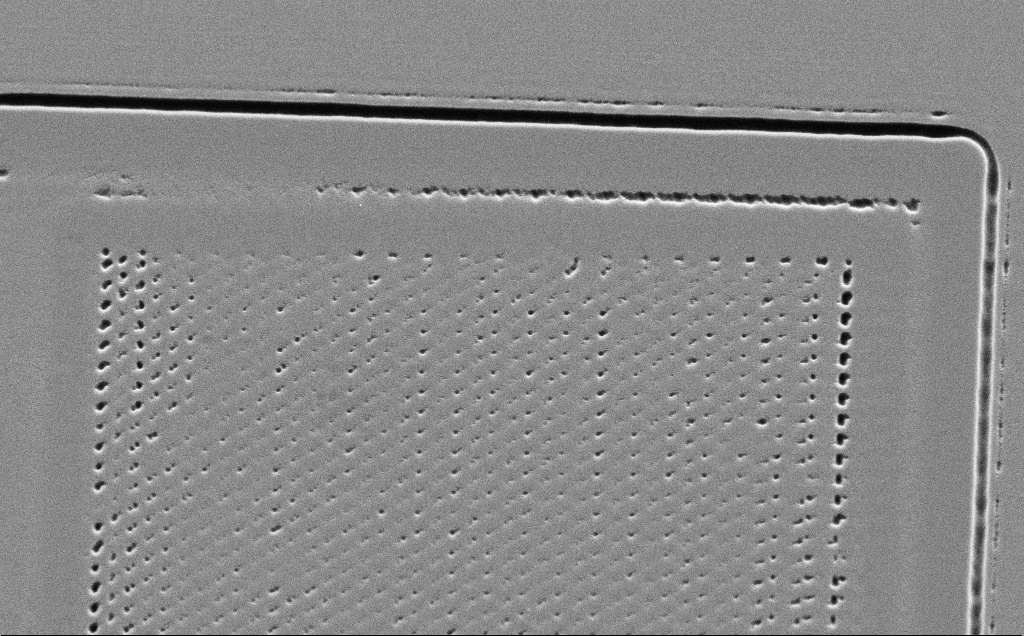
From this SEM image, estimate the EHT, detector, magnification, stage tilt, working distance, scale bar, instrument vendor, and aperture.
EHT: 5 kV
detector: SE2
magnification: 3.76 K X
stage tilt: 45°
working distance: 10 mm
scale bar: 10000 nm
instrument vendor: Zeiss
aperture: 30 µm